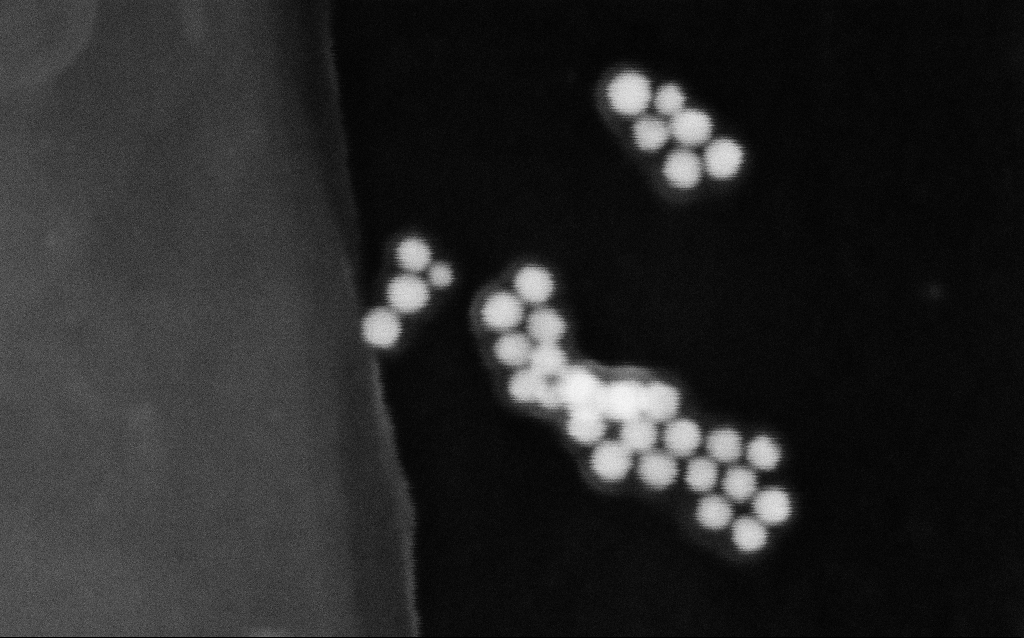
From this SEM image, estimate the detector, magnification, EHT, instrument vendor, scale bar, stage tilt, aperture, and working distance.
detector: SE2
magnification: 725.28 K X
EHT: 30 kV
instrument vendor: Zeiss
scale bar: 20 nm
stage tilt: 0°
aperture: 30 µm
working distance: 12.9 mm